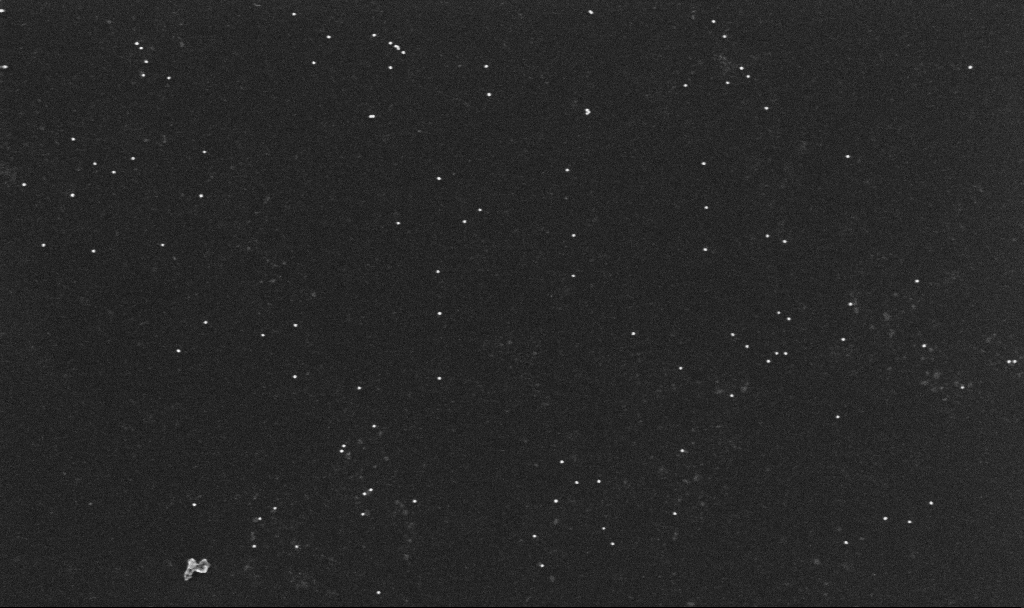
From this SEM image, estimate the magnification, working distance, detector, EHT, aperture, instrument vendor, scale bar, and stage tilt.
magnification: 100 K X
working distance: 3.2 mm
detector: InLens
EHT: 10 kV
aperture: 30 µm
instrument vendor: Zeiss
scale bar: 200 nm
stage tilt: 0°